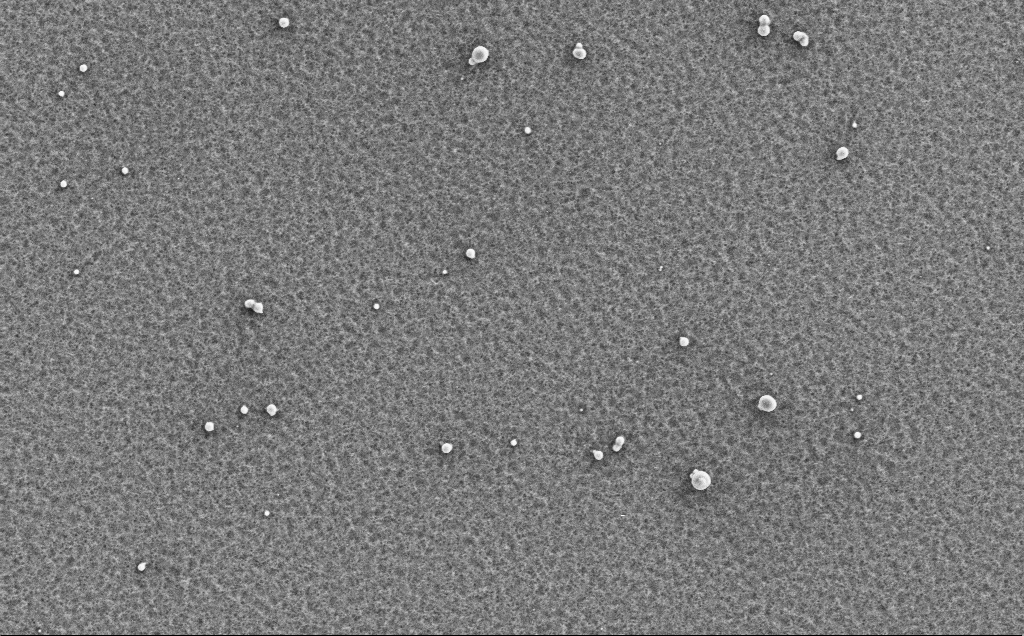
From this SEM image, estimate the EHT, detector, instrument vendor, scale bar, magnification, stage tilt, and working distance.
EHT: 5 kV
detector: SE2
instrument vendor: Zeiss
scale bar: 10000 nm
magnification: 4.14 K X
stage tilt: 0°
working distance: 5 mm